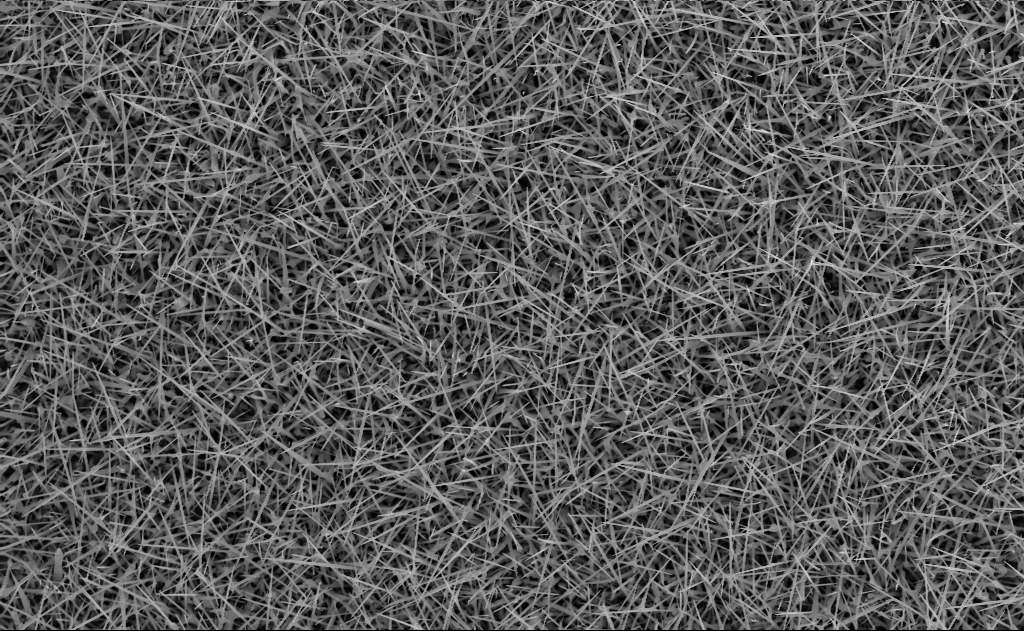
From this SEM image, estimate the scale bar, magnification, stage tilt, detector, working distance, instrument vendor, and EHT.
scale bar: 2000 nm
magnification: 10 K X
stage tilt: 0°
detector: InLens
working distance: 10 mm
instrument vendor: Zeiss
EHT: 10 kV